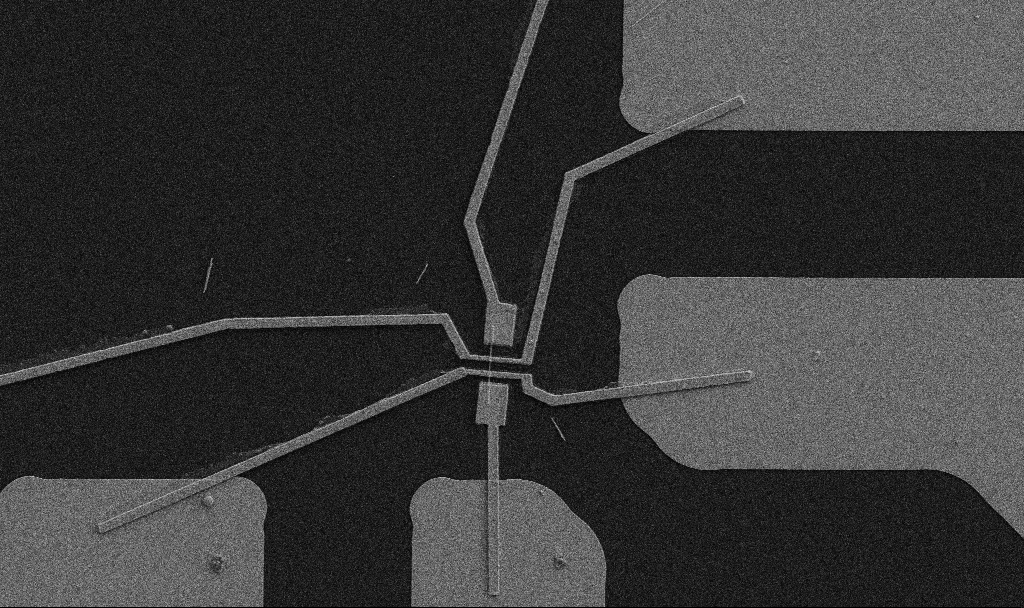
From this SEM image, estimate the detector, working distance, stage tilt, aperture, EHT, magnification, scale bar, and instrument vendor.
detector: SE2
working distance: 10.7 mm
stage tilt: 0°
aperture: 30 µm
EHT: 5 kV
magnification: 5 K X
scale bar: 10000 nm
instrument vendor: Zeiss